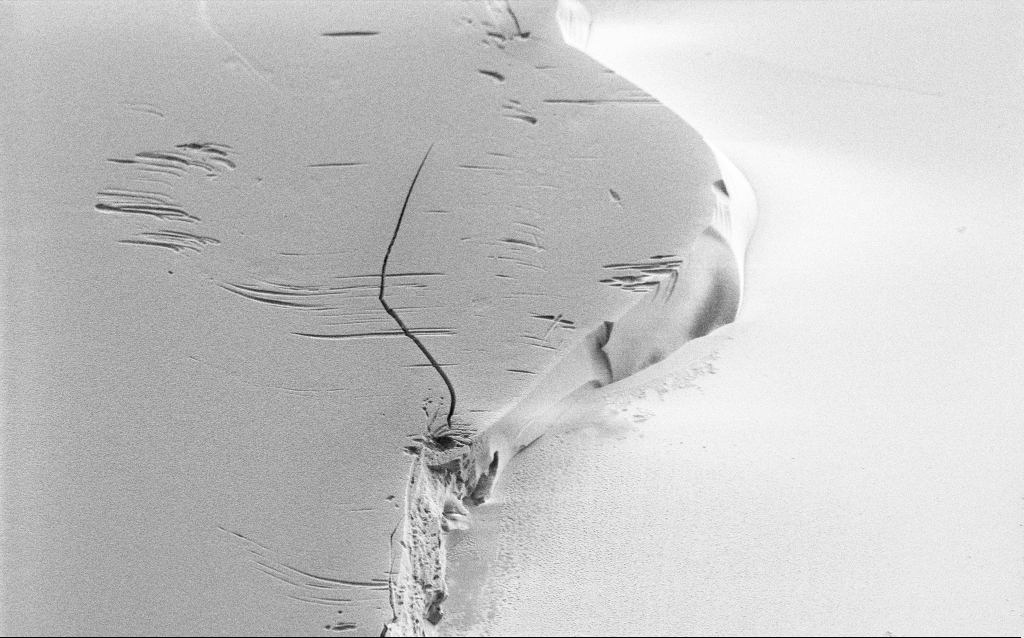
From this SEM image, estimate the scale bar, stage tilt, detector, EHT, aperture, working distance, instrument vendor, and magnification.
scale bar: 10000 nm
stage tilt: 45°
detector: SE2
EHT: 1.2 kV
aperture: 30 µm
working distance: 8 mm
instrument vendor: Zeiss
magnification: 4.82 K X